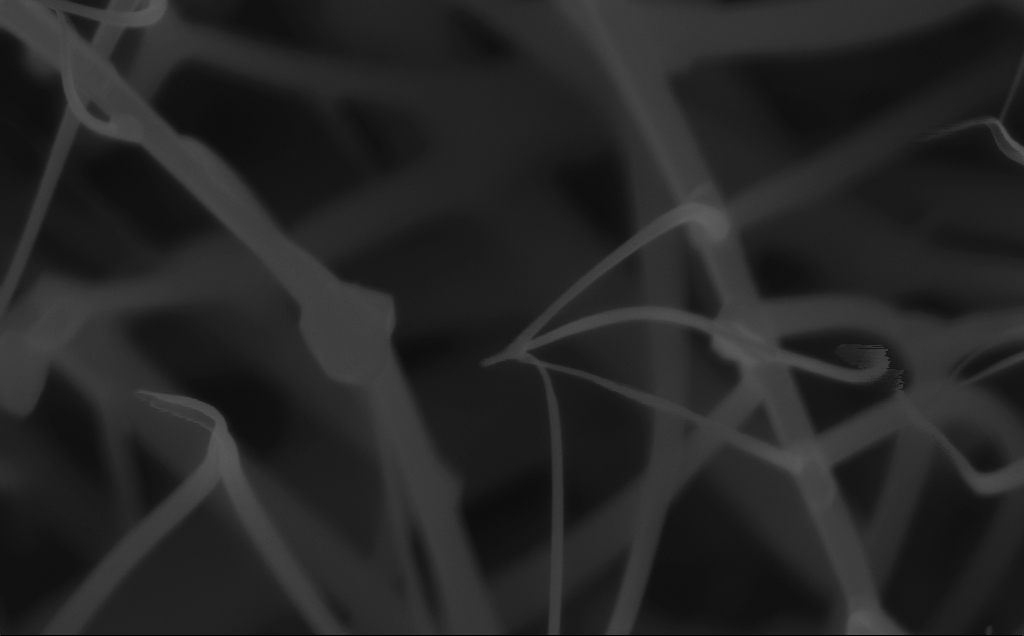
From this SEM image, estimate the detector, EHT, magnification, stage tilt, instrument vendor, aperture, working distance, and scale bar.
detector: InLens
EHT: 10 kV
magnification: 150 K X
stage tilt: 0°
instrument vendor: Zeiss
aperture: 30 µm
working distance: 6 mm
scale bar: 100 nm